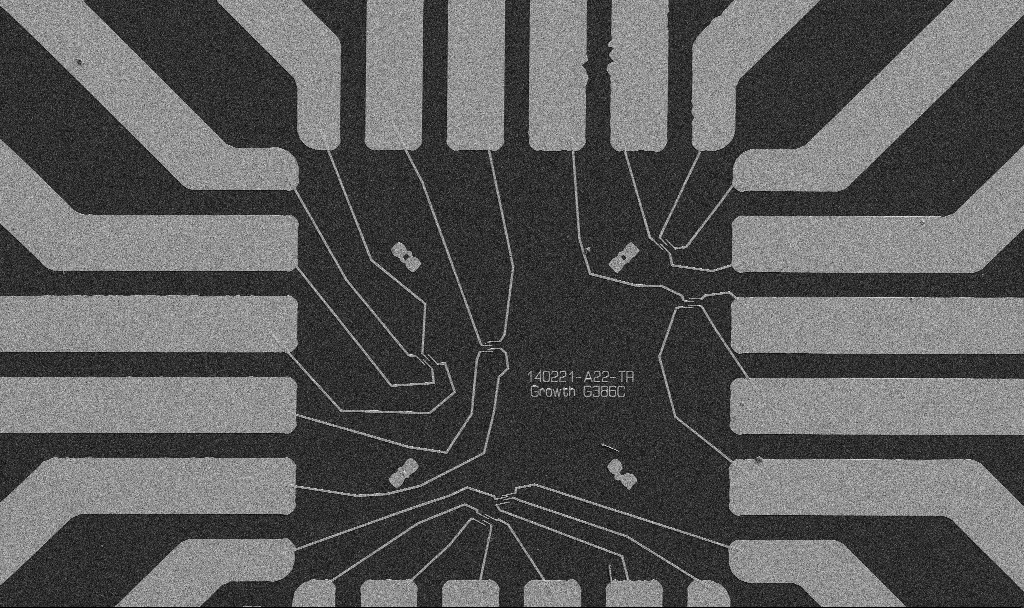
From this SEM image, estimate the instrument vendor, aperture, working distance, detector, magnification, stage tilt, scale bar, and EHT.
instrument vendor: Zeiss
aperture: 30 µm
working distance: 10.7 mm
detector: SE2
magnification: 1 K X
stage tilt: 0°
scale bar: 20000 nm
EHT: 5 kV